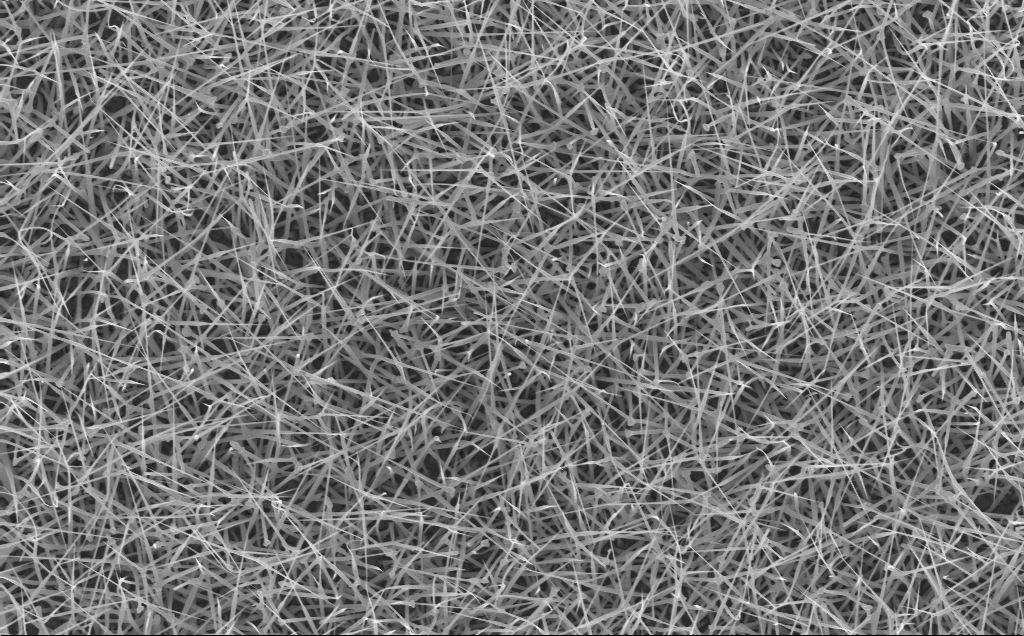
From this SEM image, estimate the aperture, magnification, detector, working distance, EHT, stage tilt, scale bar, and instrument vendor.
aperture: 30 µm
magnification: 10 K X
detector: InLens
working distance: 6 mm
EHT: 10 kV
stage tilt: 0°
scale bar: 2000 nm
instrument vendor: Zeiss